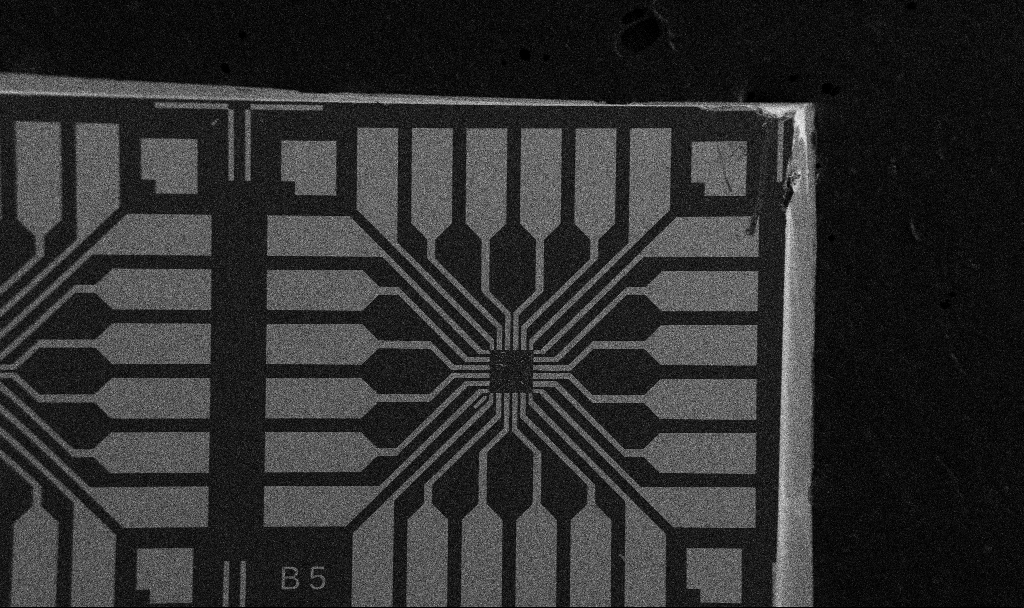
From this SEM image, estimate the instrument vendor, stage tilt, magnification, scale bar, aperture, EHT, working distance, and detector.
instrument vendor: Zeiss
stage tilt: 0°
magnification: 0.1 K X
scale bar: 200000 nm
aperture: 30 µm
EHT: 5 kV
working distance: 10.7 mm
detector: SE2